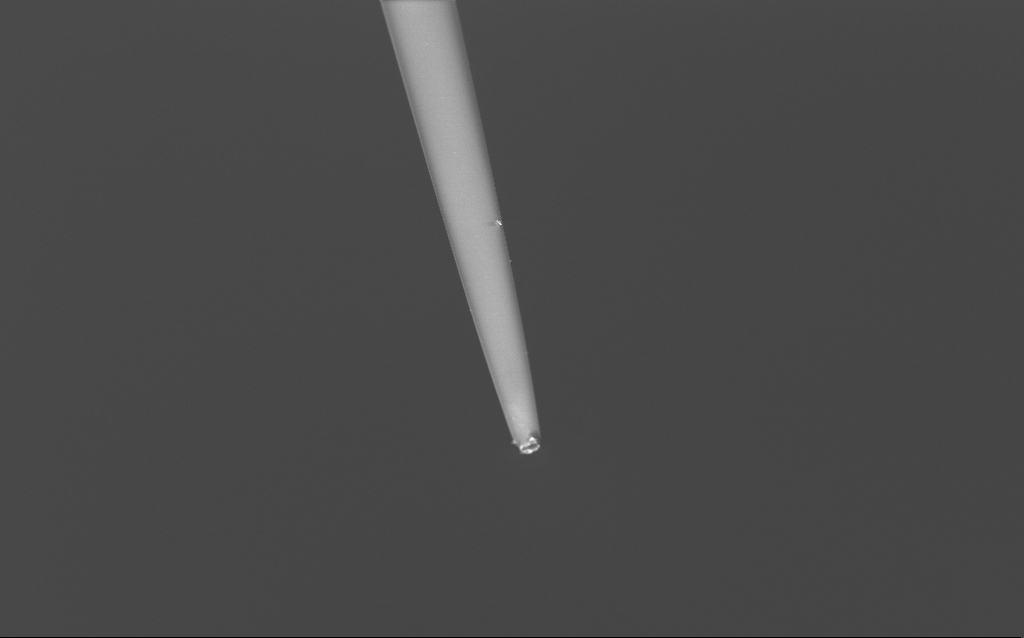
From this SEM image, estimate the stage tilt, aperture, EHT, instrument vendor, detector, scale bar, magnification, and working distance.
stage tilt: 45°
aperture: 30 µm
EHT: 2 kV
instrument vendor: Zeiss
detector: InLens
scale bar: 20000 nm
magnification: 1 K X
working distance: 6 mm